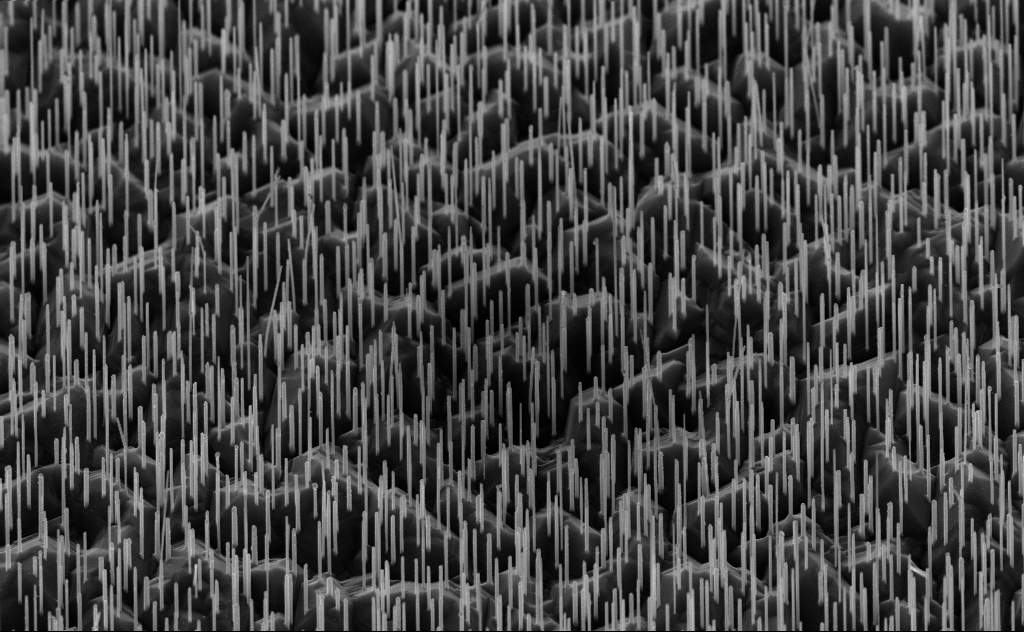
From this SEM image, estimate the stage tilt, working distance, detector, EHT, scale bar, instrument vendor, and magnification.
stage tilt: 45°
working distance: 6 mm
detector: InLens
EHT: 10 kV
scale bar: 1000 nm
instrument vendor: Zeiss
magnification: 20 K X